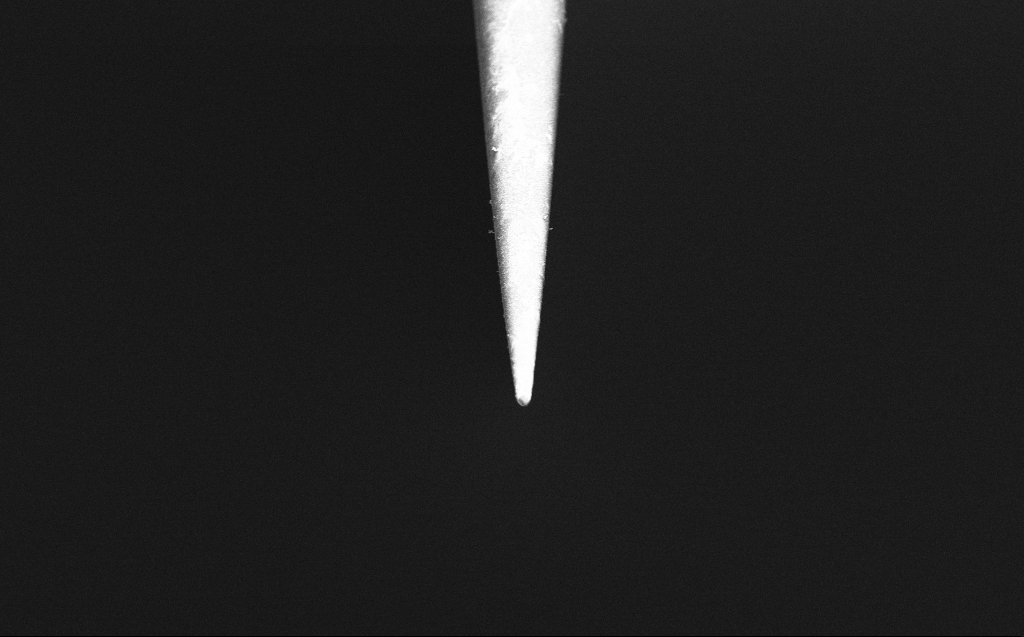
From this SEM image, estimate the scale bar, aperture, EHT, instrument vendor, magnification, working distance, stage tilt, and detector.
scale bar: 2000 nm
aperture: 30 µm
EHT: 2 kV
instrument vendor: Zeiss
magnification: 10 K X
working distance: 4 mm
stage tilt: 45°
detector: InLens